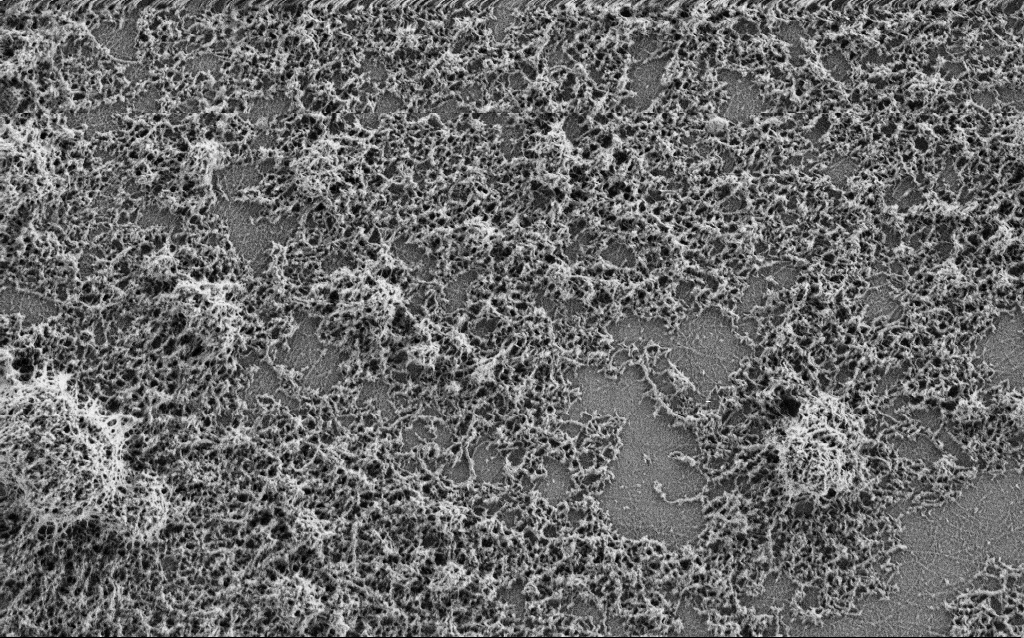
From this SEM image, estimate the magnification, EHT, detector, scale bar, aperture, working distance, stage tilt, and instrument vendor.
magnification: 15 K X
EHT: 0.9 kV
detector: SE2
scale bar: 2000 nm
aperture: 30 µm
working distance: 4 mm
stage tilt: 0°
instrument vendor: Zeiss